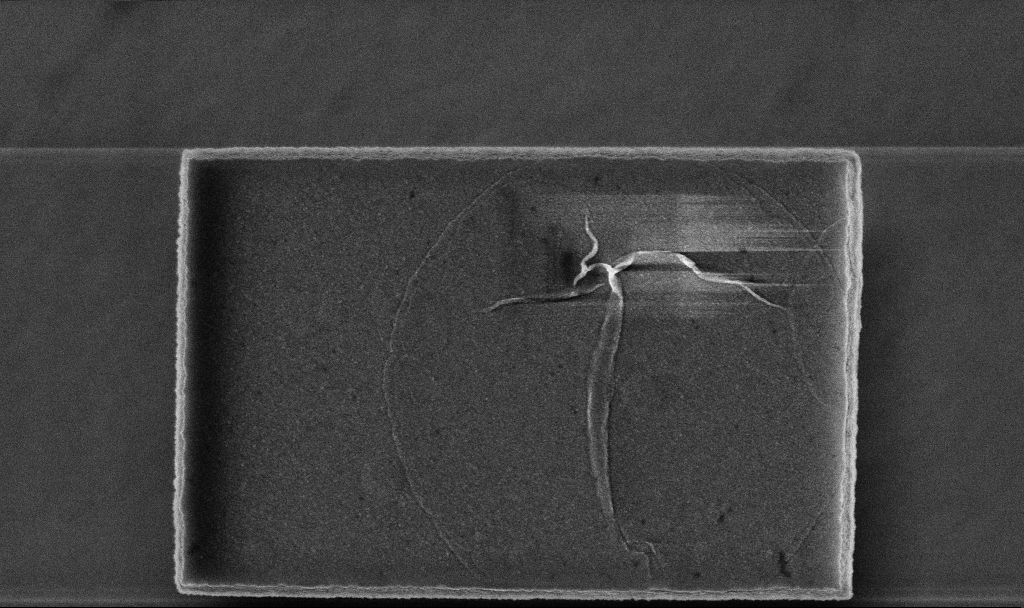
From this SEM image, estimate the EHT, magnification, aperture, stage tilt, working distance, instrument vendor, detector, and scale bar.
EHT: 5 kV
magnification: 54.76 K X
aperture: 30 µm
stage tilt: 0°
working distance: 3.2 mm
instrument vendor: Zeiss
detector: InLens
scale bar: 1000 nm